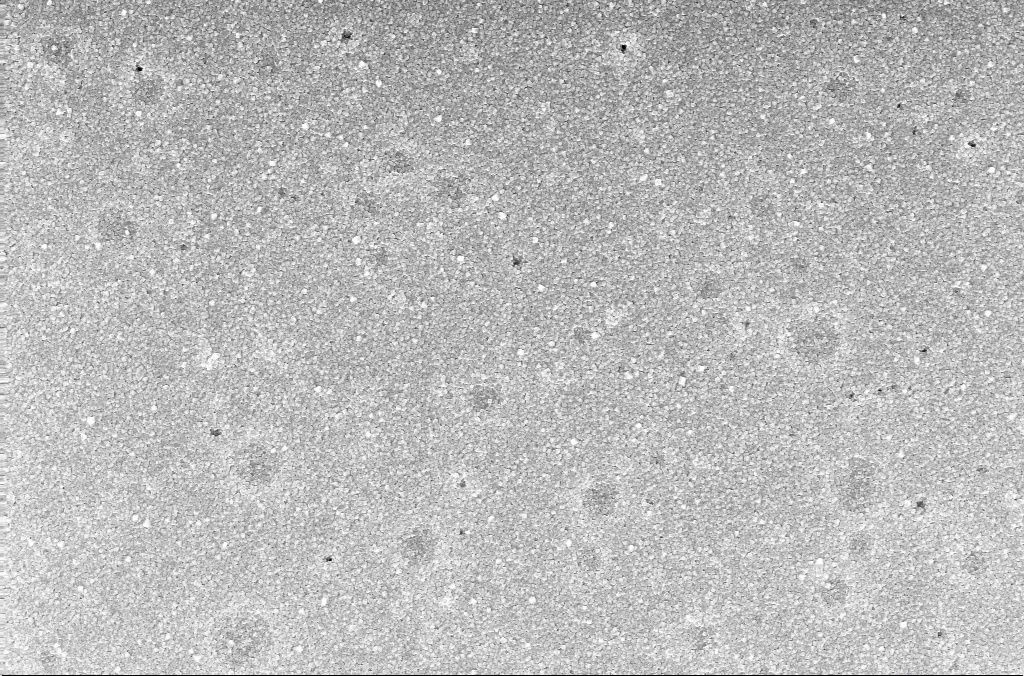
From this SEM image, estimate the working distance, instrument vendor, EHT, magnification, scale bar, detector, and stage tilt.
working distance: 3.9 mm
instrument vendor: Zeiss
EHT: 20 kV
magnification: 10 K X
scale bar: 2000 nm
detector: InLens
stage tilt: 0°